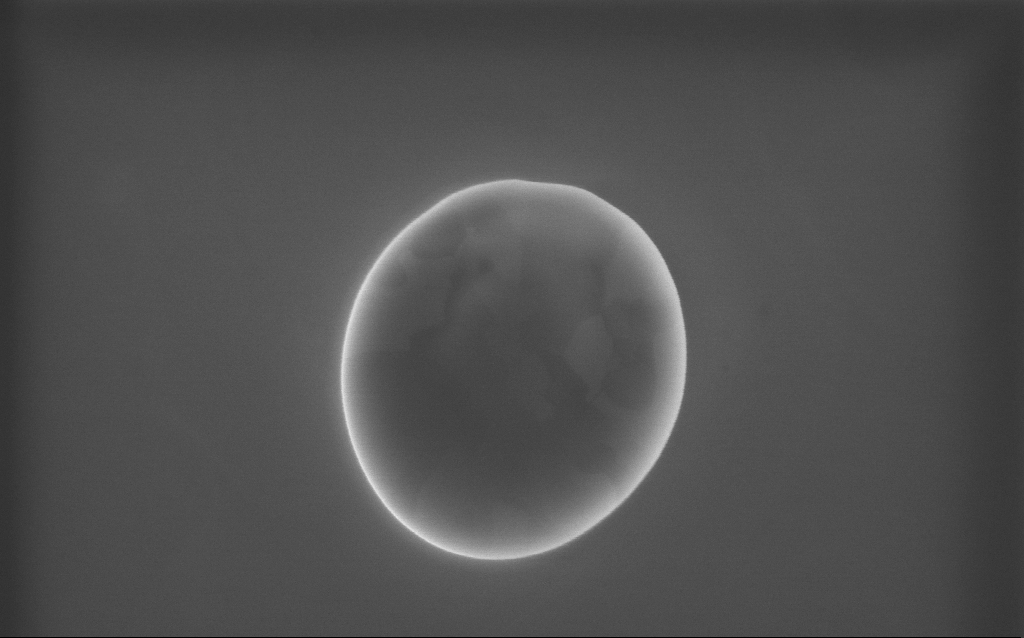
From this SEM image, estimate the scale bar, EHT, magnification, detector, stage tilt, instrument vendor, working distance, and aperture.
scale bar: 1000 nm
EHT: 10 kV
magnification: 70 K X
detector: InLens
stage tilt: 0°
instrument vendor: Zeiss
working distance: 2 mm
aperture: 30 µm